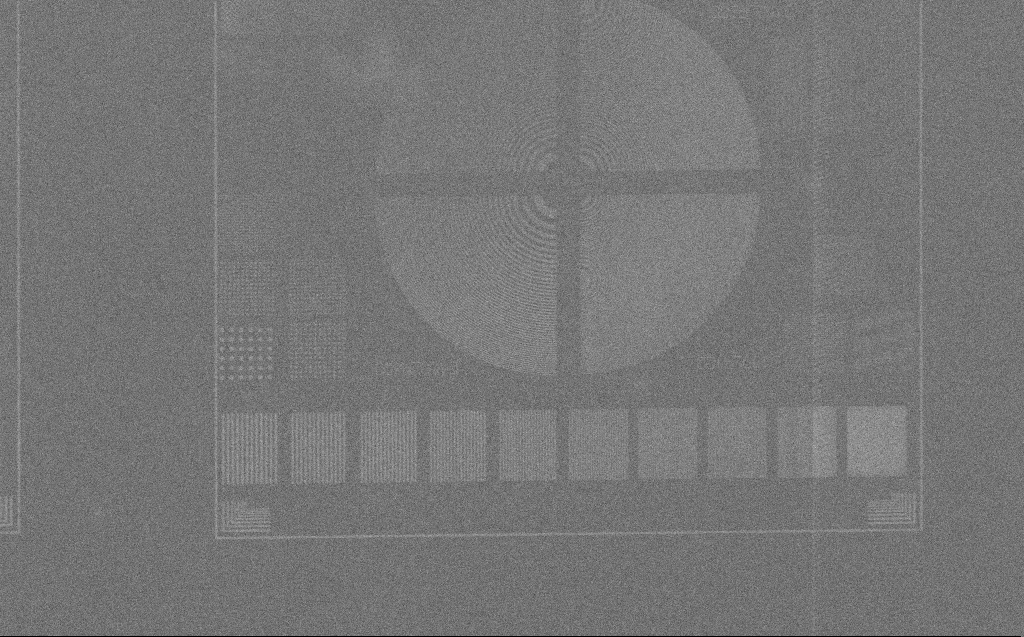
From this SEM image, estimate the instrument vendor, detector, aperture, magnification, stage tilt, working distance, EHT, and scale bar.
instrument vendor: Zeiss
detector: SE2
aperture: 30 µm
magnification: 0.886 K X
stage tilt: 0°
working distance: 4 mm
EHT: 3 kV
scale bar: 20000 nm